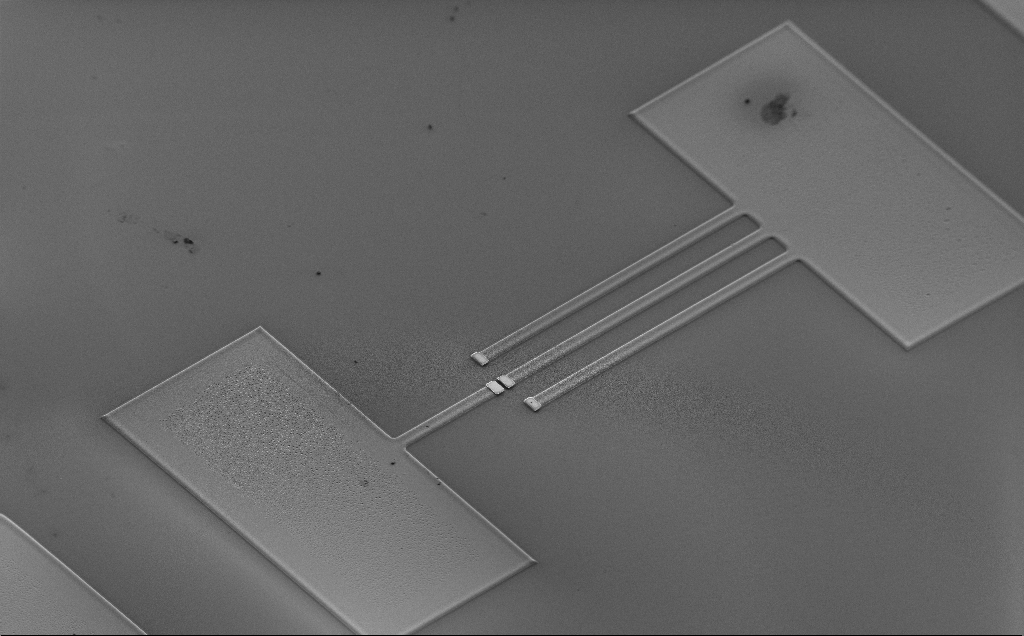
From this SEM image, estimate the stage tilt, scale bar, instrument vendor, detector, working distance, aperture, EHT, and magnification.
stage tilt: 43°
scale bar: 100000 nm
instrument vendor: Zeiss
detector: SE2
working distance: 10 mm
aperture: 30 µm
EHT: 2 kV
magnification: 0.38 K X